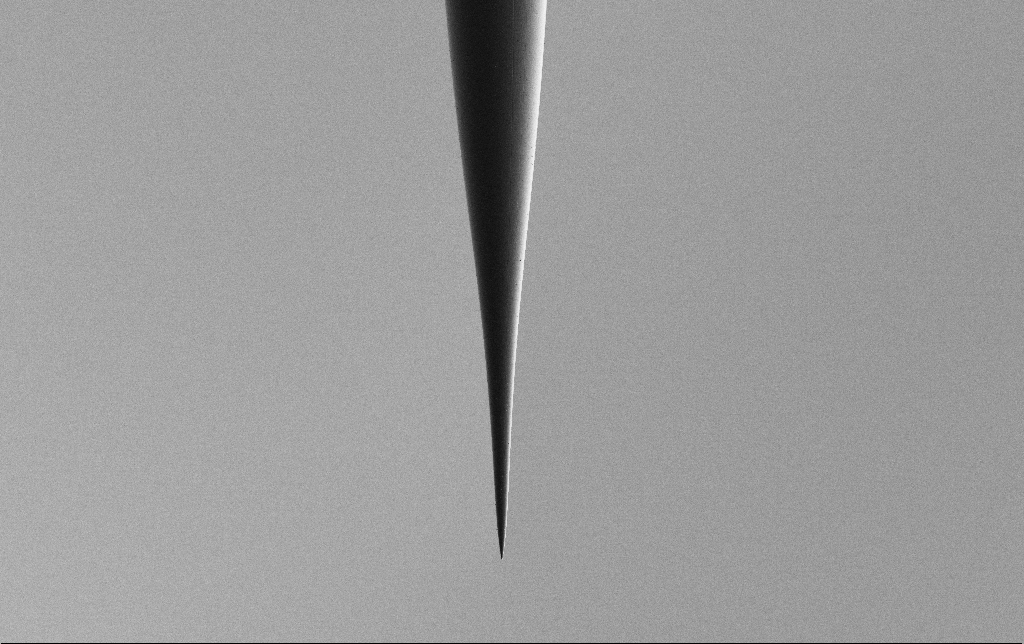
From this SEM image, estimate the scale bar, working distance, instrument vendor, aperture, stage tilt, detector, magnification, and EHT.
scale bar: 100000 nm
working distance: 6.5 mm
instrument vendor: Zeiss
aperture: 30 µm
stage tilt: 0°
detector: SE2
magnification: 0.5 K X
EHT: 2 kV